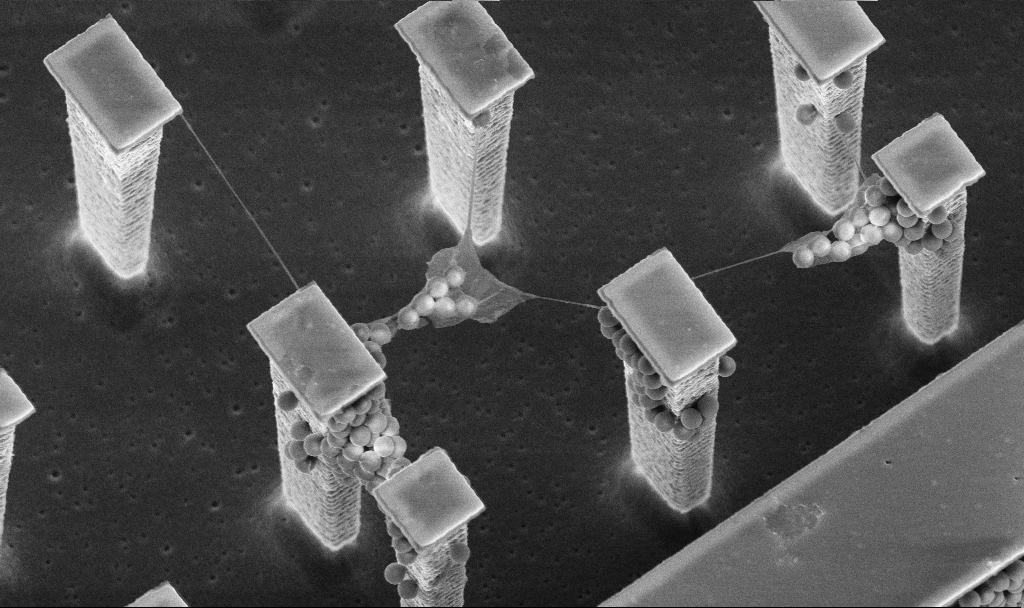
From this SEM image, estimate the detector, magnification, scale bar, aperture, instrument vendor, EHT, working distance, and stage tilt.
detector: InLens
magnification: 10.73 K X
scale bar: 2000 nm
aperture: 30 µm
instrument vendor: Zeiss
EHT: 5 kV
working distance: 4.8 mm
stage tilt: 20°